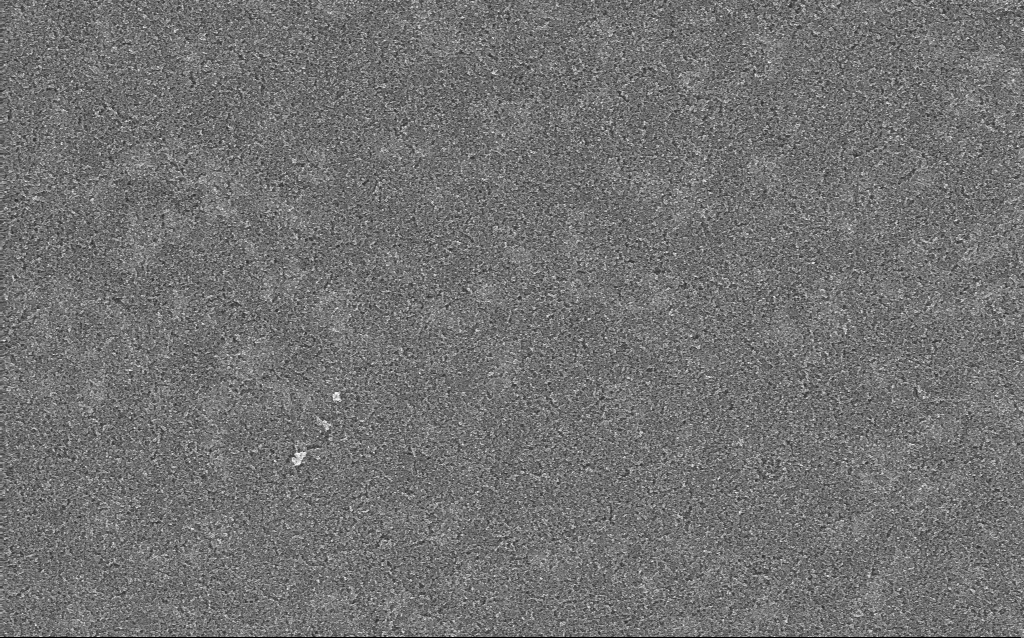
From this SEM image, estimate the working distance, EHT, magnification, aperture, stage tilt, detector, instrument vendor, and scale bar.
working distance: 5 mm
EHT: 10 kV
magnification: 23.9 K X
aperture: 30 µm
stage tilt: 0°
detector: InLens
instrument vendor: Zeiss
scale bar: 1000 nm